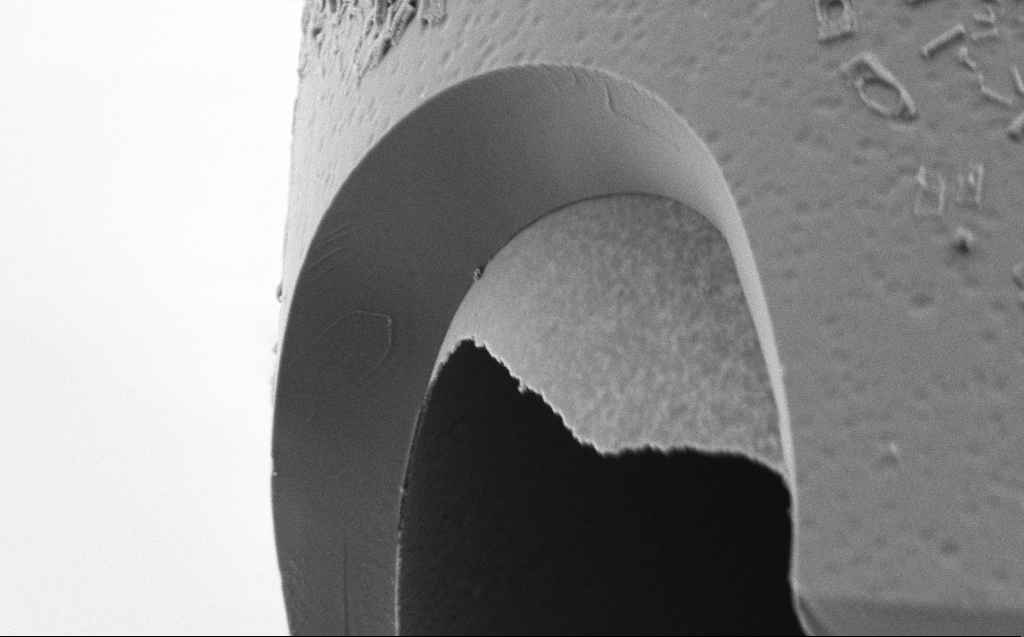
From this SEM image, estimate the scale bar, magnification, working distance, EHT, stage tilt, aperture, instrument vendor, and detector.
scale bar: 1000 nm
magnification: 24.52 K X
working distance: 4 mm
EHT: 2 kV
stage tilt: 45°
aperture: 30 µm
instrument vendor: Zeiss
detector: SE2